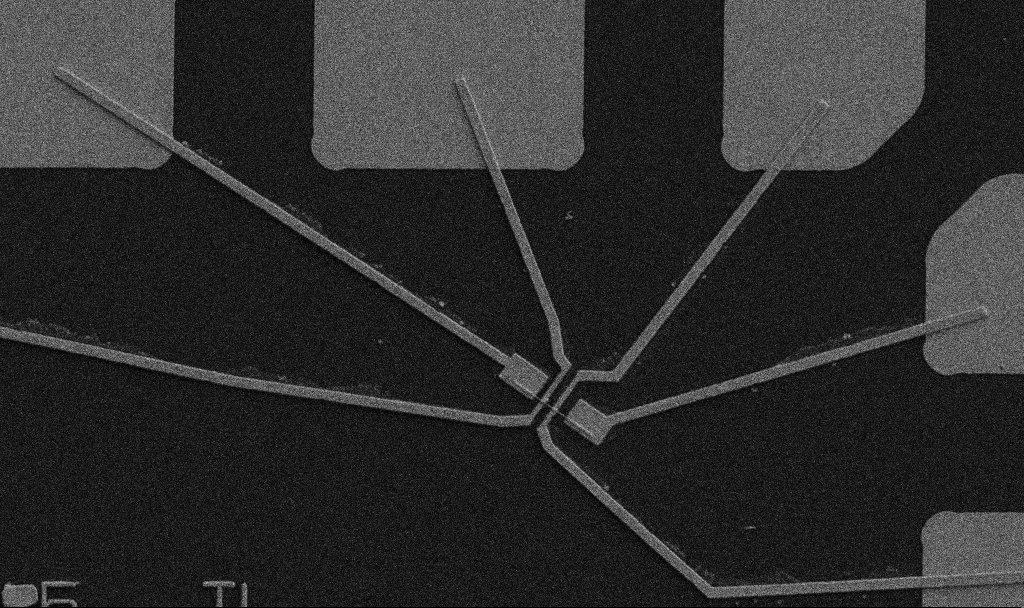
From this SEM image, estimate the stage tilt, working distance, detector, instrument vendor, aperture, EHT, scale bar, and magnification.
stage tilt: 0°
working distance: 10.7 mm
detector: SE2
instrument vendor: Zeiss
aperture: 30 µm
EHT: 5 kV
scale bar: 10000 nm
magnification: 5 K X